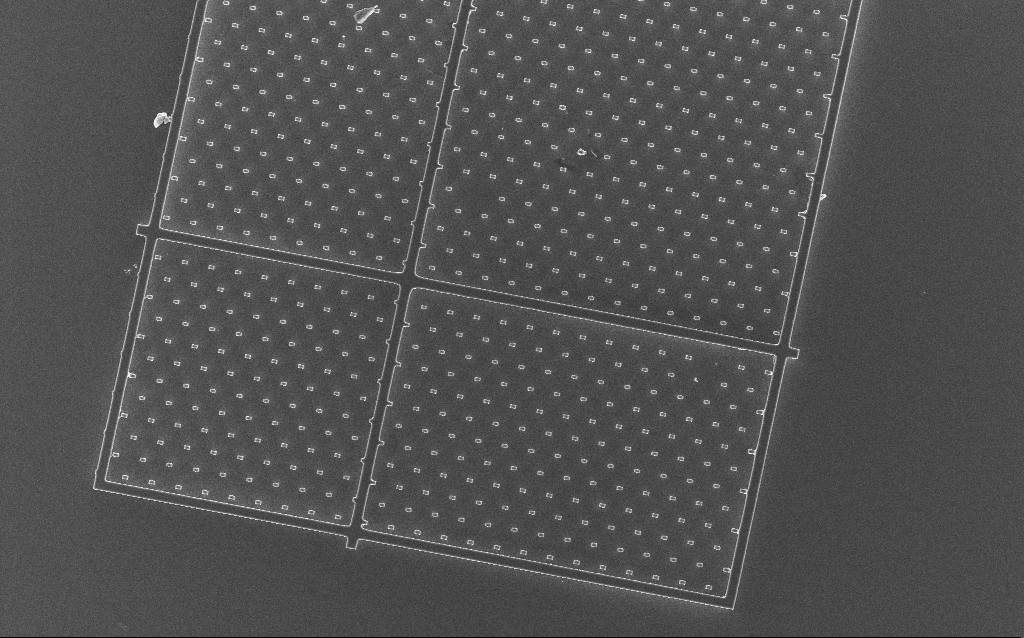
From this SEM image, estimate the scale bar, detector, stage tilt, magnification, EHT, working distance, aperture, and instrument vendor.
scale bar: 20000 nm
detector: InLens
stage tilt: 0°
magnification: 0.825 K X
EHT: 10 kV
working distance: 5.4 mm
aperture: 30 µm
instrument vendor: Zeiss